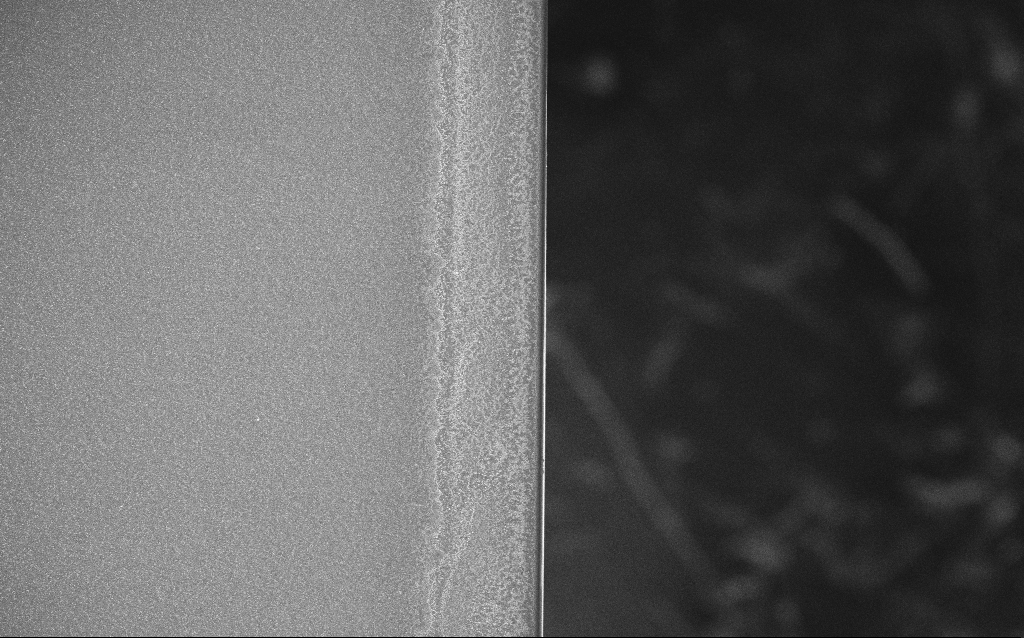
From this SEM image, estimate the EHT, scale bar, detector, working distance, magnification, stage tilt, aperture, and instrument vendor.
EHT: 20 kV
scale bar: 100000 nm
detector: InLens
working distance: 1.8 mm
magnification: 0.25 K X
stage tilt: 0°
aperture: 30 µm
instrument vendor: Zeiss